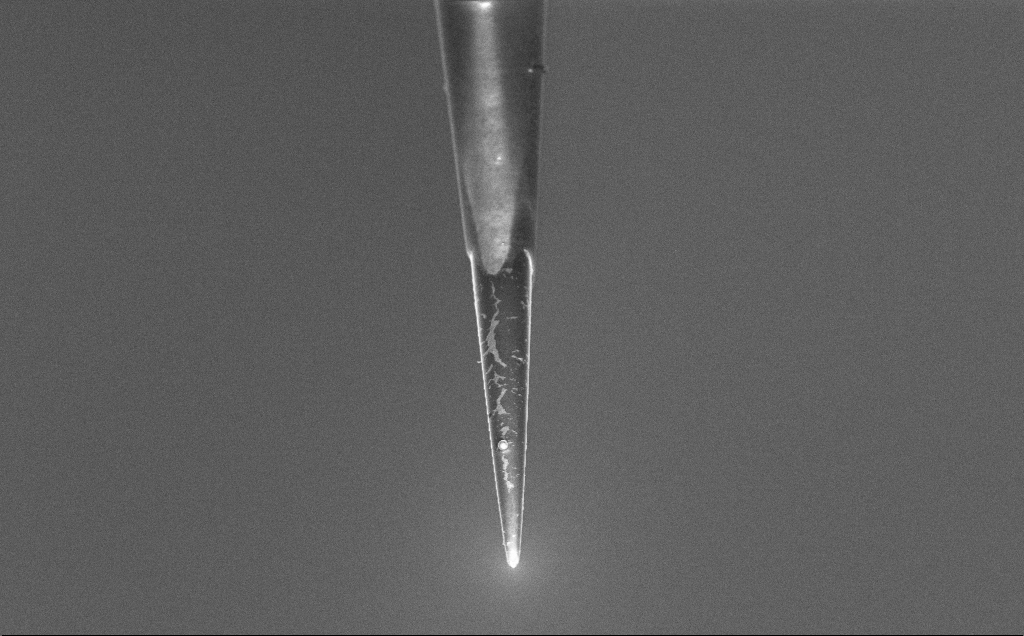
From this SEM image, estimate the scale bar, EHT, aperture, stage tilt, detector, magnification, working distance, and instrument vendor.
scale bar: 2000 nm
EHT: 3 kV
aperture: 30 µm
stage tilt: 45°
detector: InLens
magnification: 7.5 K X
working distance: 7.5 mm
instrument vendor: Zeiss